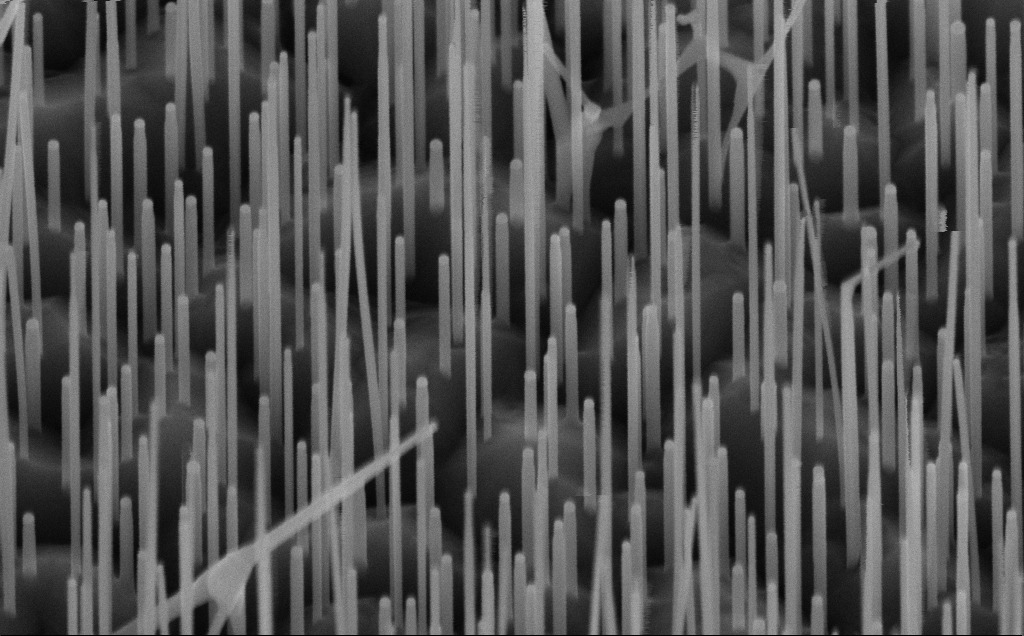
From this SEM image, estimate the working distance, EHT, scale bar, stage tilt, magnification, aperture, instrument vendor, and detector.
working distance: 7 mm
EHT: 10 kV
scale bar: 200 nm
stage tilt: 45°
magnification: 100 K X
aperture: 30 µm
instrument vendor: Zeiss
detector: InLens